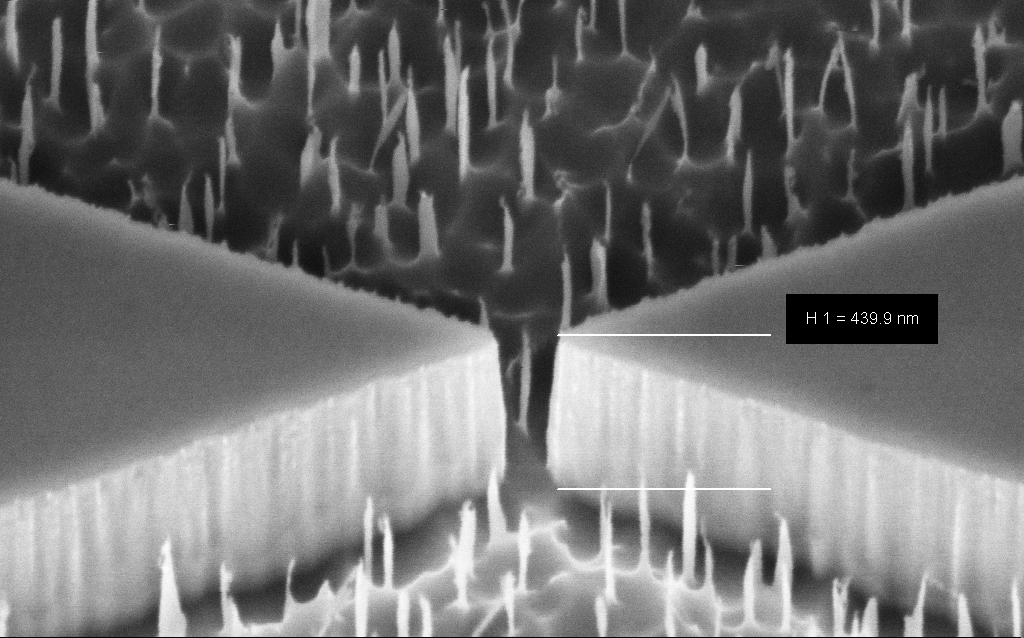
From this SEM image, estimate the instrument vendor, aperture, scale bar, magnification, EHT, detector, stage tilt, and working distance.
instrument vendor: Zeiss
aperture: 30 µm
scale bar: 200 nm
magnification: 128.53 K X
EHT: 3 kV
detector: InLens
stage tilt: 45°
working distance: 8 mm